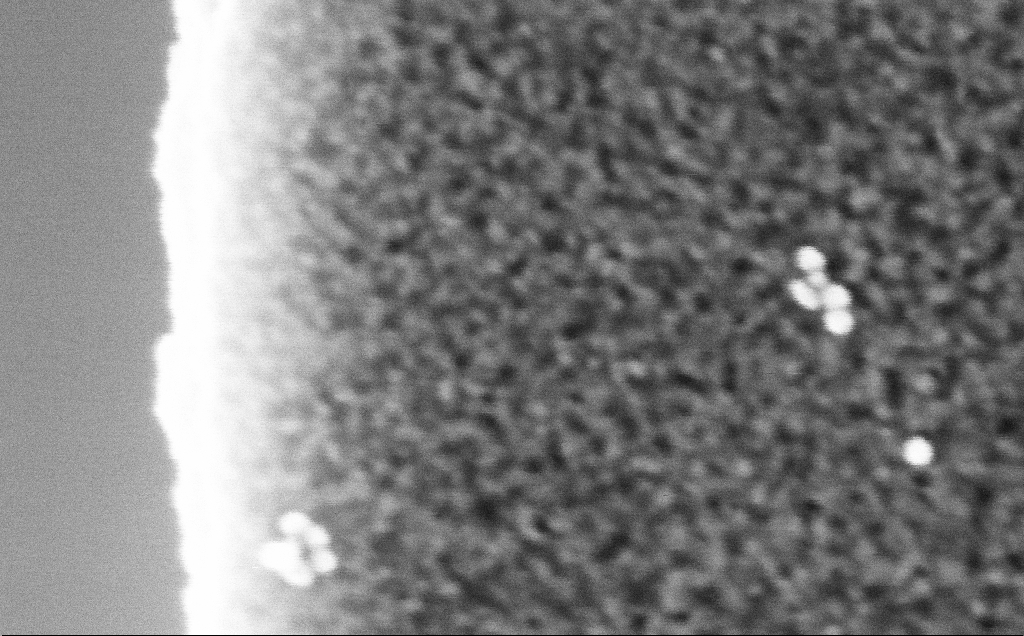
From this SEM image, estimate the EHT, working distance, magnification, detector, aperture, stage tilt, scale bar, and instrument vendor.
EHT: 3 kV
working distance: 2.5 mm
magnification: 581.98 K X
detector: InLens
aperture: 30 µm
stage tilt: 0°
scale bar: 100 nm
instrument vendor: Zeiss